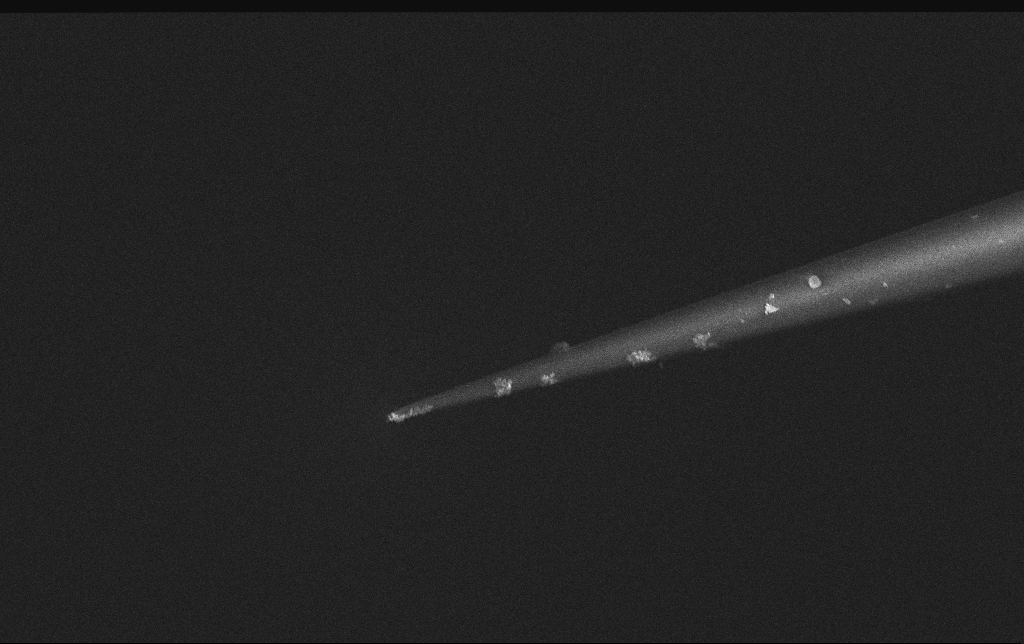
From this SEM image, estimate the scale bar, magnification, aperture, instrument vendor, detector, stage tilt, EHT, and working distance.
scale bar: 1000 nm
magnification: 25 K X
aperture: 30 µm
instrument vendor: Zeiss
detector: InLens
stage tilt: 0°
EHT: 3 kV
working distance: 6.2 mm